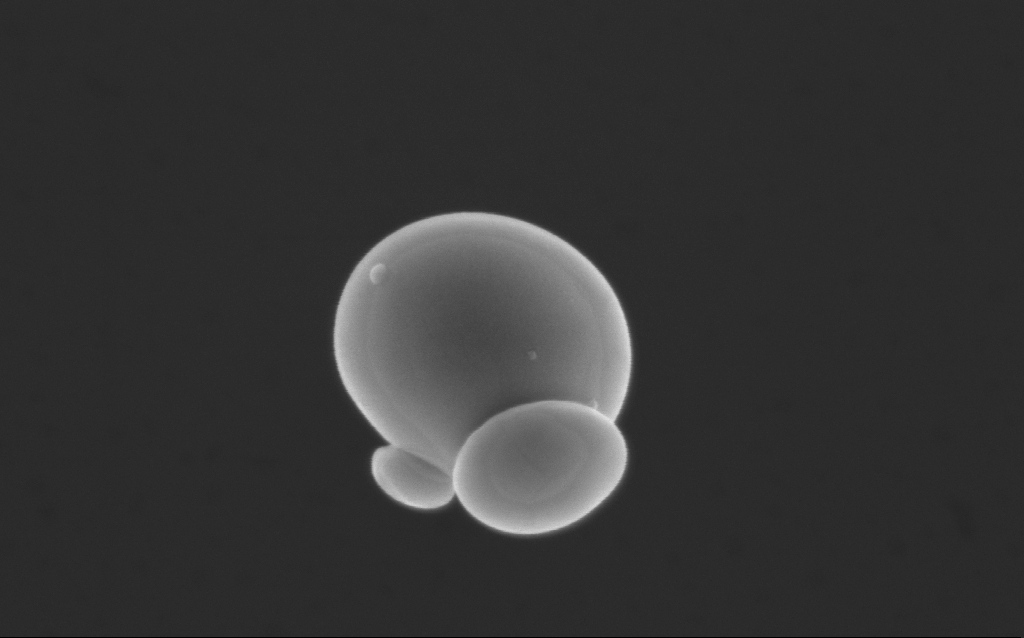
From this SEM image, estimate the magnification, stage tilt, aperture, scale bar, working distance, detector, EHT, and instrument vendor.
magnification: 130.83 K X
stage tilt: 0°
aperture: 30 µm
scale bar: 200 nm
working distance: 4 mm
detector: InLens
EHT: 10 kV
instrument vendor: Zeiss